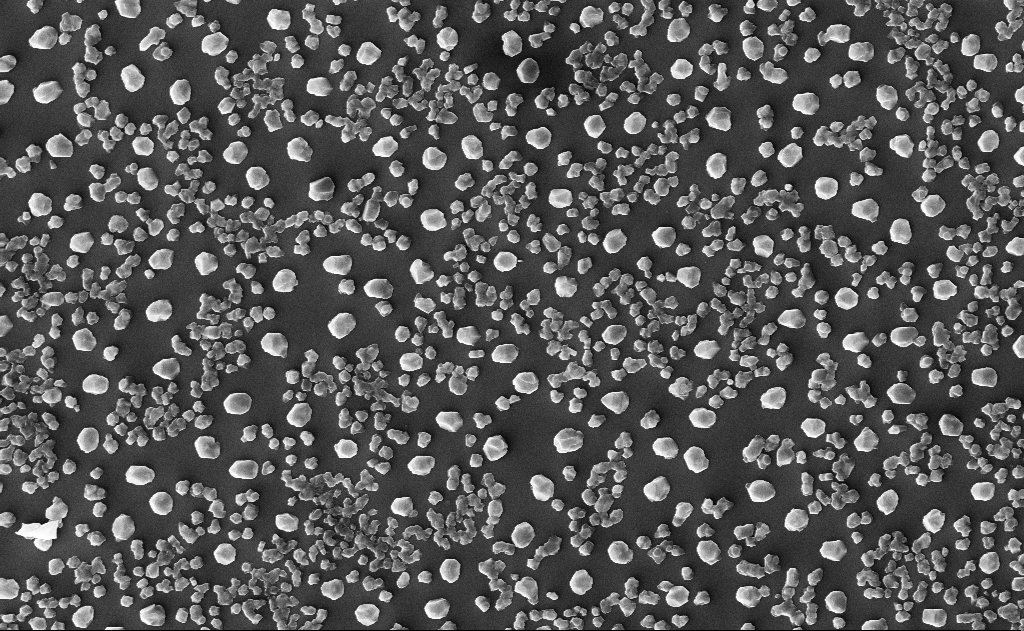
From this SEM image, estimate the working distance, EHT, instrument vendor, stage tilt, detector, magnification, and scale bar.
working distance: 16 mm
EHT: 10 kV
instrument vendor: Zeiss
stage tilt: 0°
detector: InLens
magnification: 20 K X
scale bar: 2000 nm